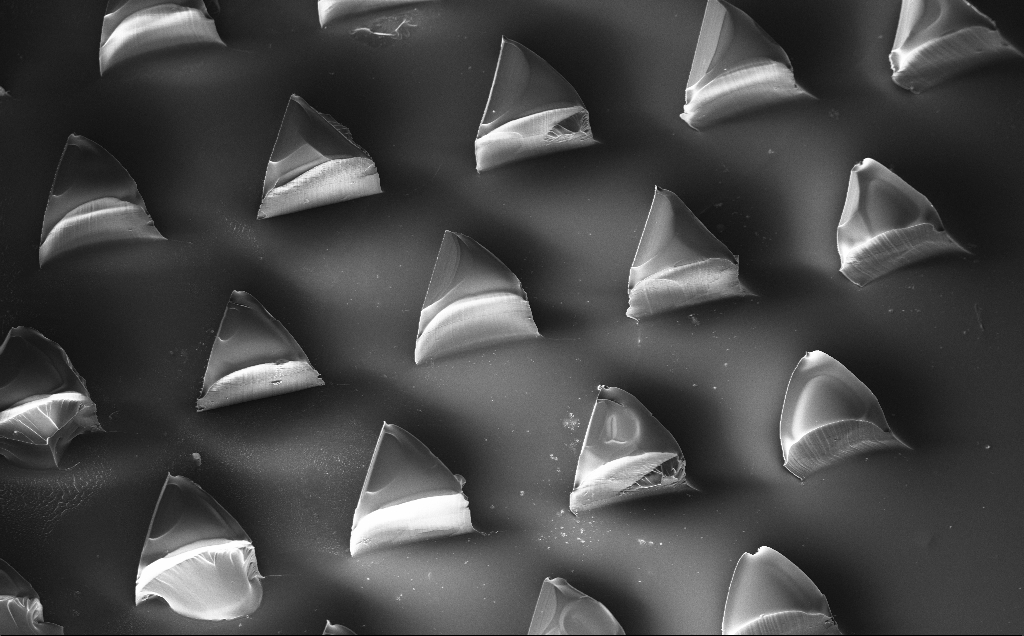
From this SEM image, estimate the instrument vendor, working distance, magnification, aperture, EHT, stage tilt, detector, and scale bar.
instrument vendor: Zeiss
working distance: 9 mm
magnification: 0.176 K X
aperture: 30 µm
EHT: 10 kV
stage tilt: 20°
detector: InLens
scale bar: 200000 nm